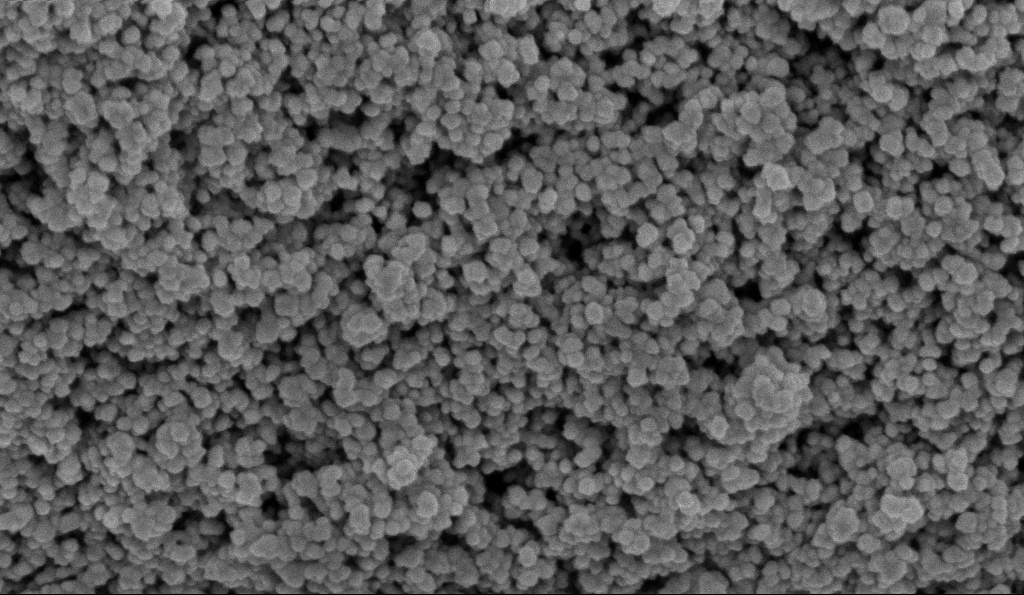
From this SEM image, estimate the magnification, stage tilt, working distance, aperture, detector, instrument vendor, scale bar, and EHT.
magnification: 135 K X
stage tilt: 0°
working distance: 5.9 mm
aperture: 30 µm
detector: InLens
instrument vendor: Zeiss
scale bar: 200 nm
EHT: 5 kV